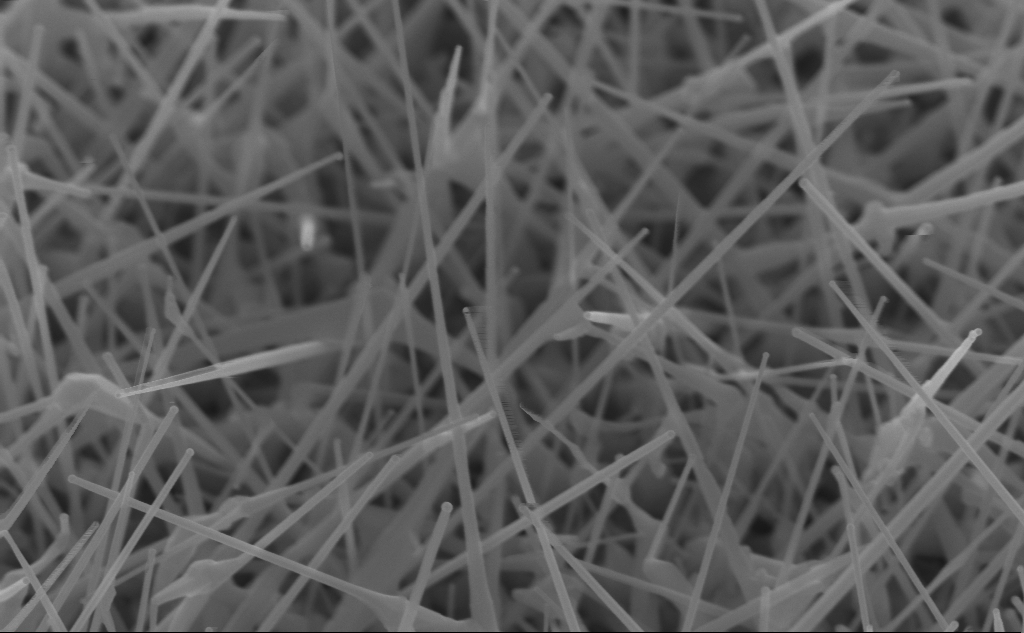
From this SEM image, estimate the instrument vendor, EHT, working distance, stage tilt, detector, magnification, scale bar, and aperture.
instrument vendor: Zeiss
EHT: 10 kV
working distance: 5 mm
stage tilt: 45°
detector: InLens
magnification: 74.21 K X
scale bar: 200 nm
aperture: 30 µm